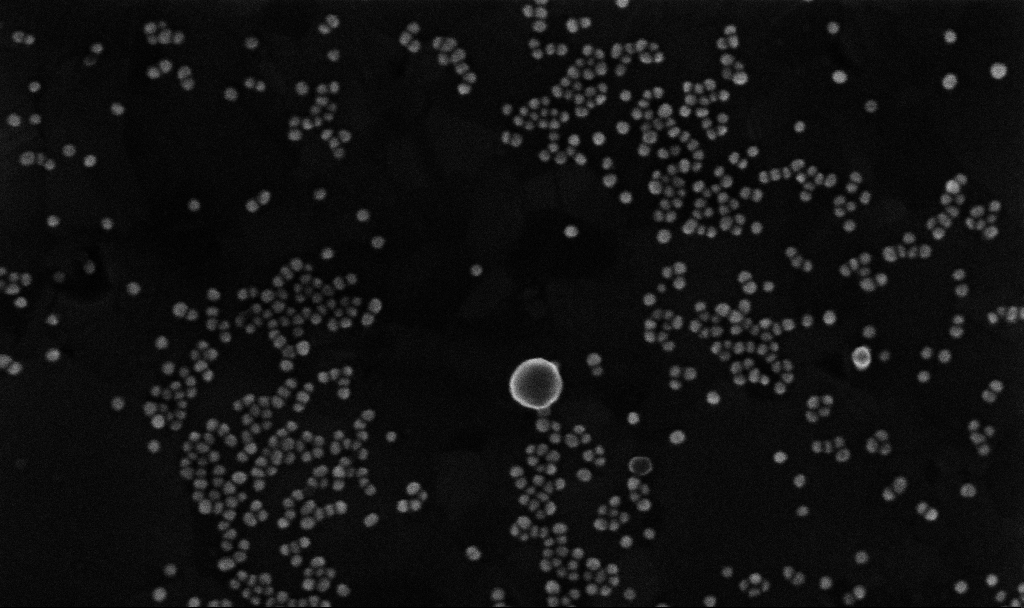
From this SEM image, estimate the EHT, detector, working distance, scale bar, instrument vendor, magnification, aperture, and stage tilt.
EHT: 10 kV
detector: InLens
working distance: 3.7 mm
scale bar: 200 nm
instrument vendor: Zeiss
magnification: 250.53 K X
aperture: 30 µm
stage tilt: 0°